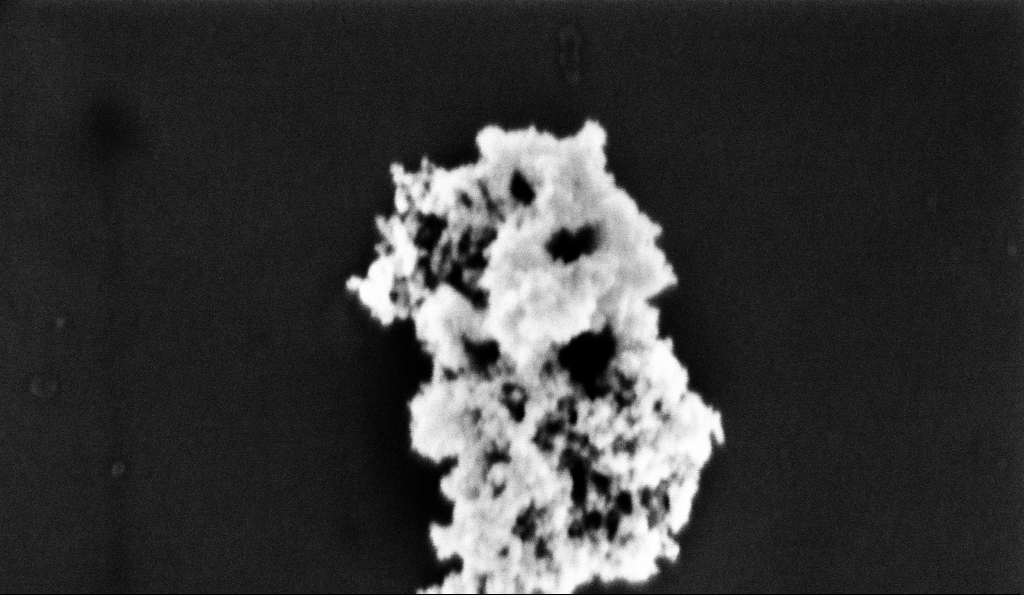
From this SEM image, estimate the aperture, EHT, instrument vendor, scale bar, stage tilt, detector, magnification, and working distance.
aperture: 30 µm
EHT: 2 kV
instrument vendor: Zeiss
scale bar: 200 nm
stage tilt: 0°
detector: InLens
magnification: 174.38 K X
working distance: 5.2 mm